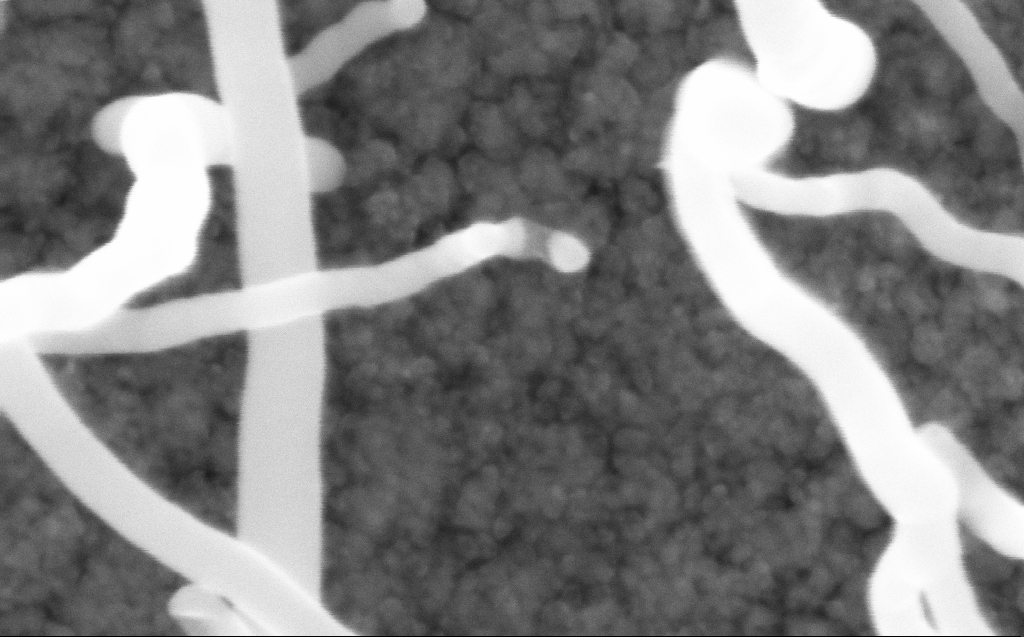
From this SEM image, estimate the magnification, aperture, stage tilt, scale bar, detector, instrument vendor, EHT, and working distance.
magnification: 400 K X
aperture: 30 µm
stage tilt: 0°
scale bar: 100 nm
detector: InLens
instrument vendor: Zeiss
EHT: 10 kV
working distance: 3 mm